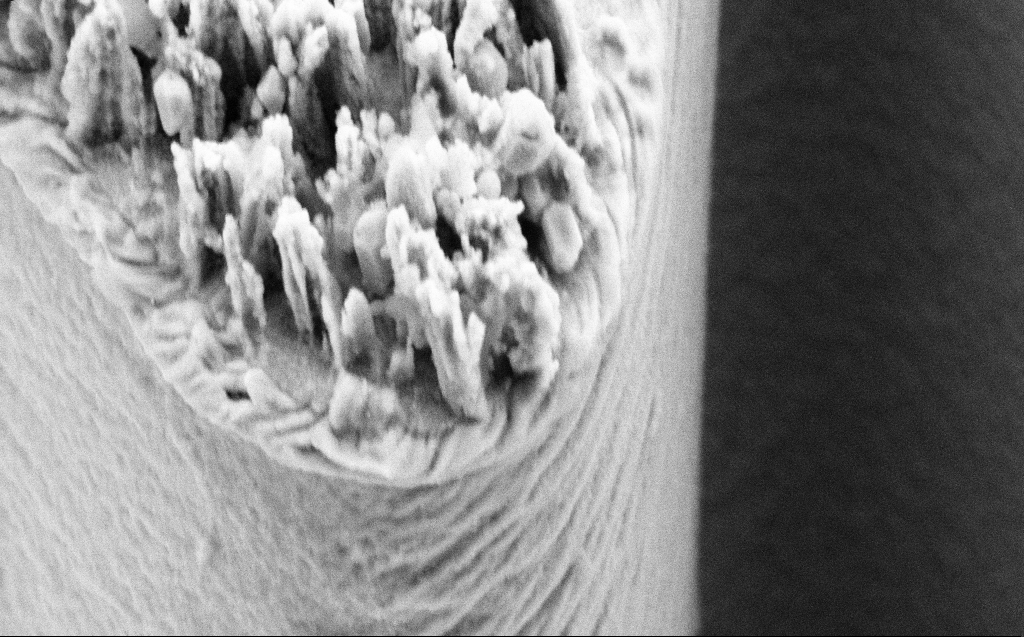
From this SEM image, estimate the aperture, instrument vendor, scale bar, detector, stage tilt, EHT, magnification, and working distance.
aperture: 30 µm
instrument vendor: Zeiss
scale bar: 1000 nm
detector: SE2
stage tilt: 45°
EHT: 5 kV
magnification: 50 K X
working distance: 9 mm